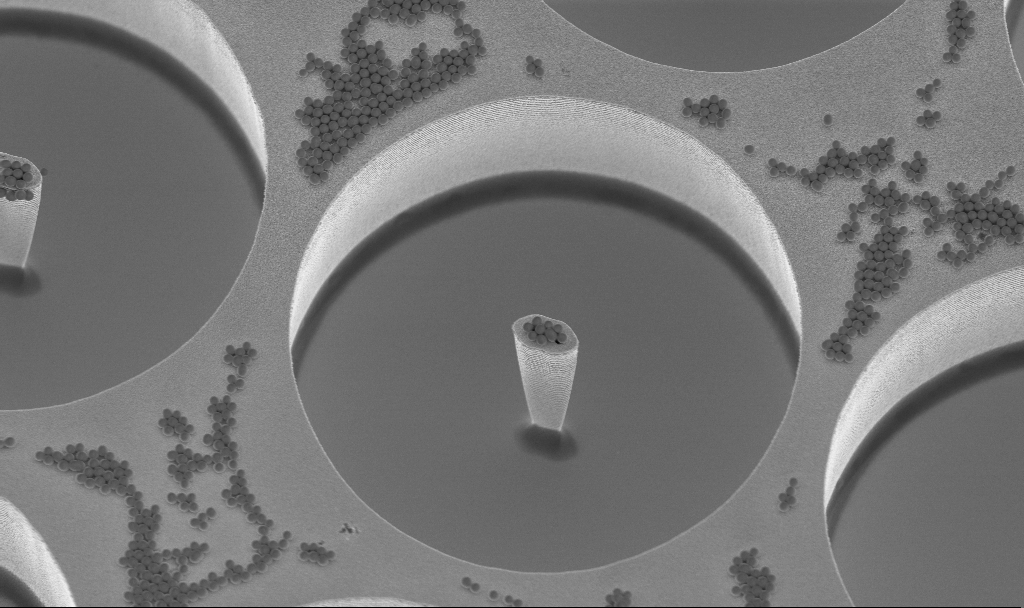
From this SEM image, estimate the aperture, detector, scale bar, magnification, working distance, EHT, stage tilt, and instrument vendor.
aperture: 30 µm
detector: InLens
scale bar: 10000 nm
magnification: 6.31 K X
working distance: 3.1 mm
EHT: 3 kV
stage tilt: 20°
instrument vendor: Zeiss